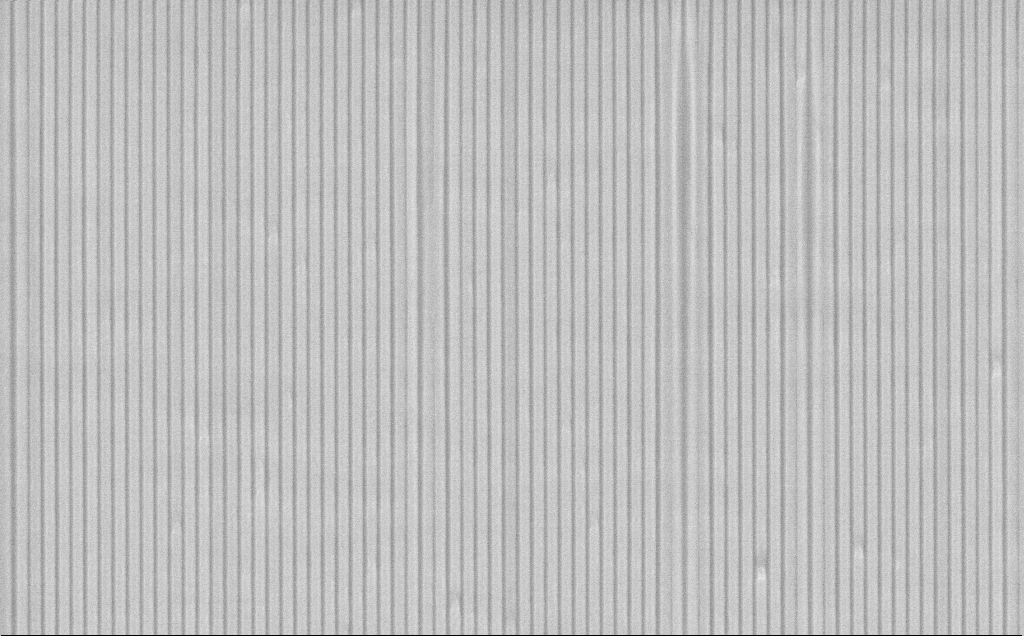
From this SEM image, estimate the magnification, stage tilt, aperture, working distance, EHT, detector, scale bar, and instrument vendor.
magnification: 28.62 K X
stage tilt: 45°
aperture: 30 µm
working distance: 8 mm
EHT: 10 kV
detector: SE2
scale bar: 1000 nm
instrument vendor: Zeiss